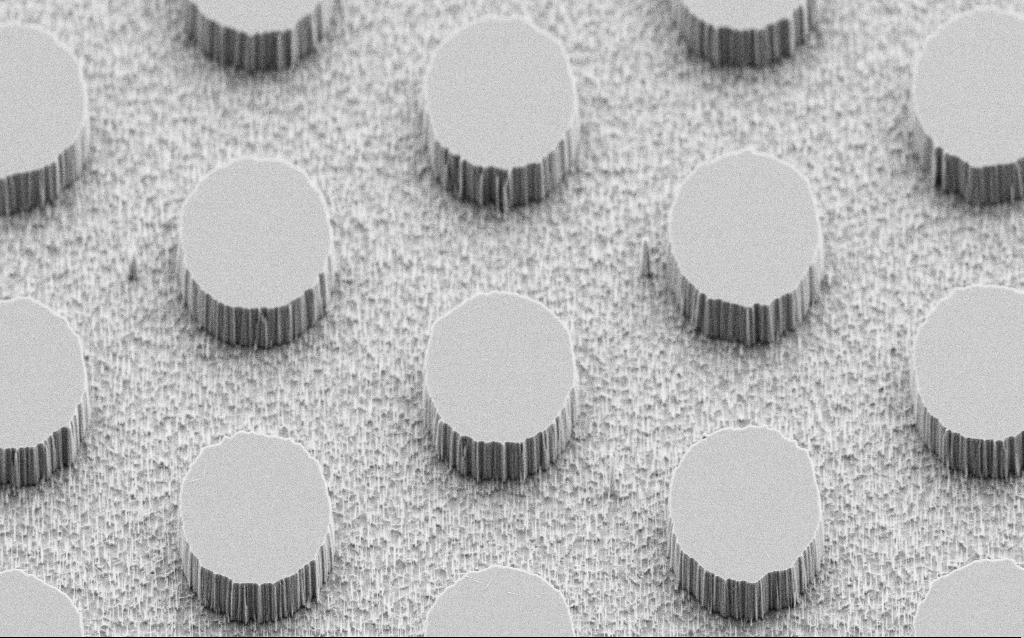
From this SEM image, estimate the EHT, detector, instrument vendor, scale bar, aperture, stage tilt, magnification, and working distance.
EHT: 5 kV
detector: SE2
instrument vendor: Zeiss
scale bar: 10000 nm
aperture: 30 µm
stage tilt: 45°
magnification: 3 K X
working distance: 7 mm